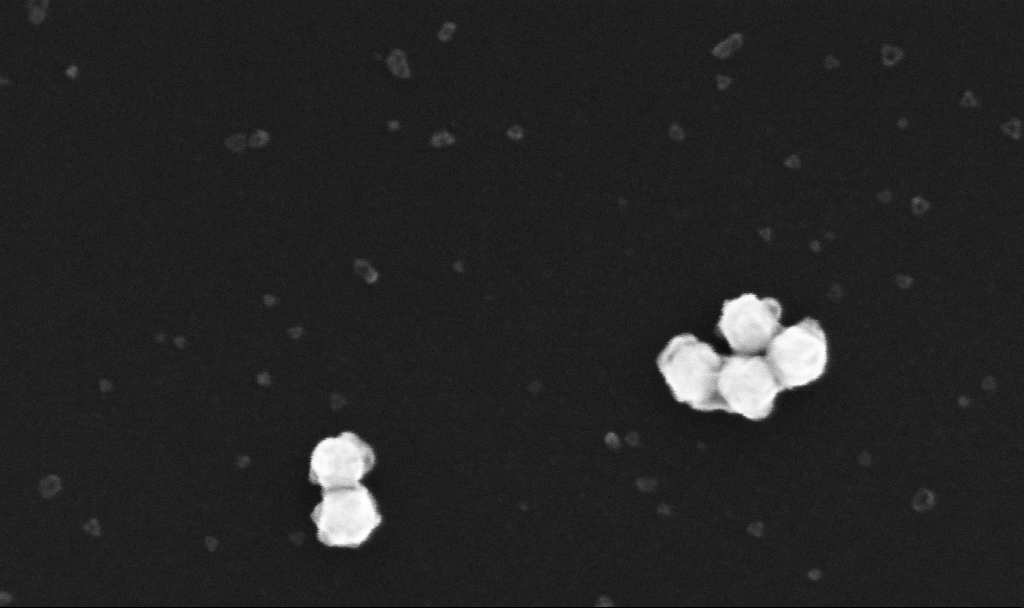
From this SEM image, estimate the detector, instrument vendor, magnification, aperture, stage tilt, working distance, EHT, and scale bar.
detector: InLens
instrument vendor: Zeiss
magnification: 350 K X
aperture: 30 µm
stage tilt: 0°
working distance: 3.4 mm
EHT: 10 kV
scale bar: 200 nm